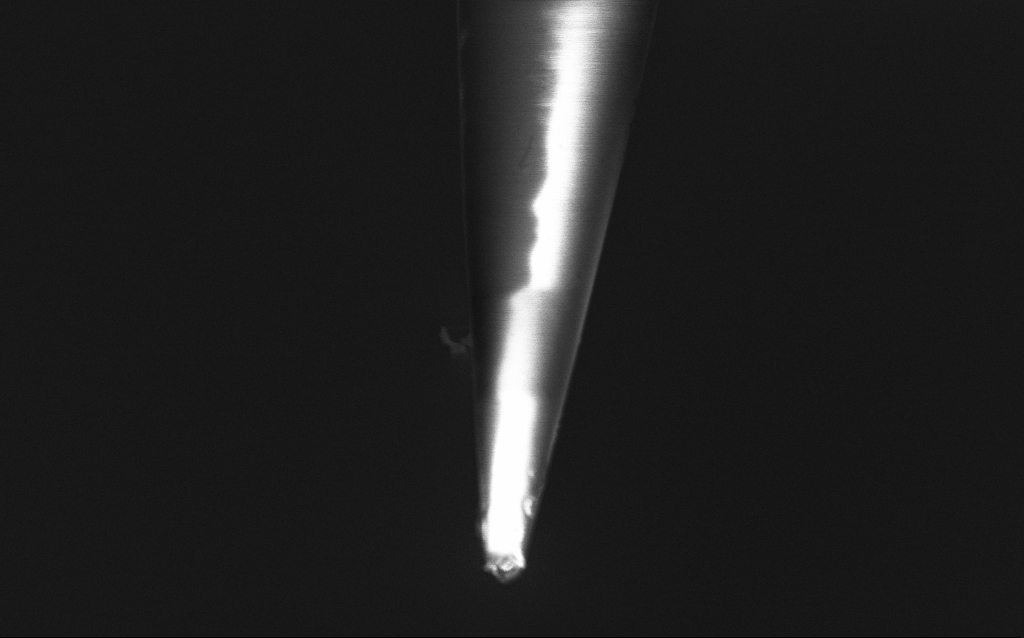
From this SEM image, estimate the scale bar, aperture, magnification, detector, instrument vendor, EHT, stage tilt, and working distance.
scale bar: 1000 nm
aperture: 30 µm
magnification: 50 K X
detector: InLens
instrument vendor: Zeiss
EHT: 2 kV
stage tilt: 45°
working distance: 6 mm